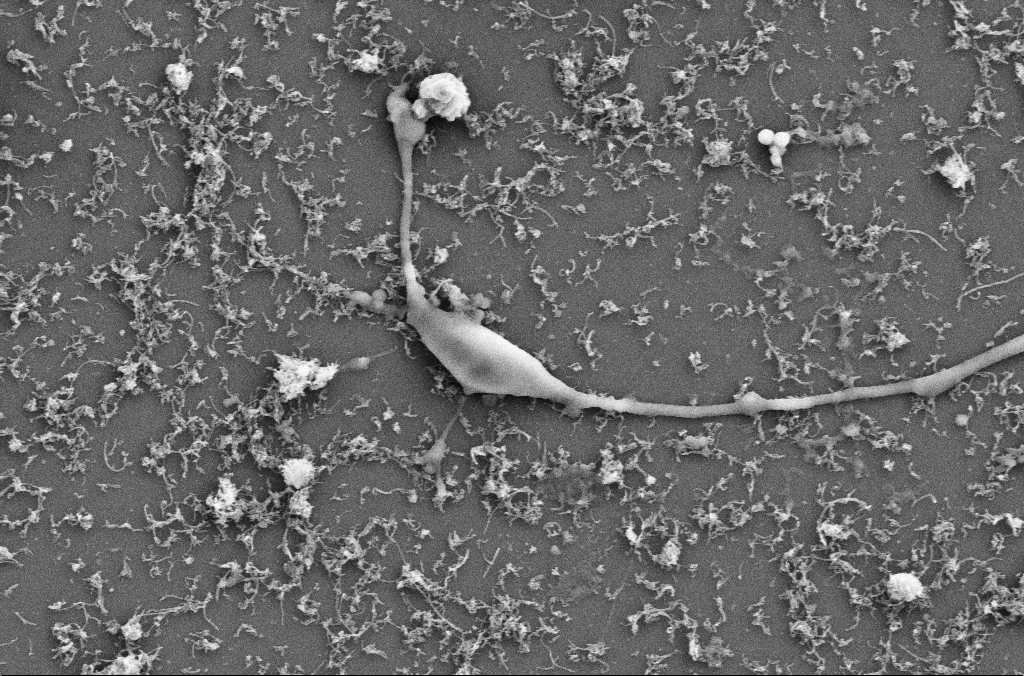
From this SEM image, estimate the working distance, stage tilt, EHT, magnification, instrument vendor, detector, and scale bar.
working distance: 4 mm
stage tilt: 0°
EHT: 5 kV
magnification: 15 K X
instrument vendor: Zeiss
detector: SE2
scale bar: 1000 nm